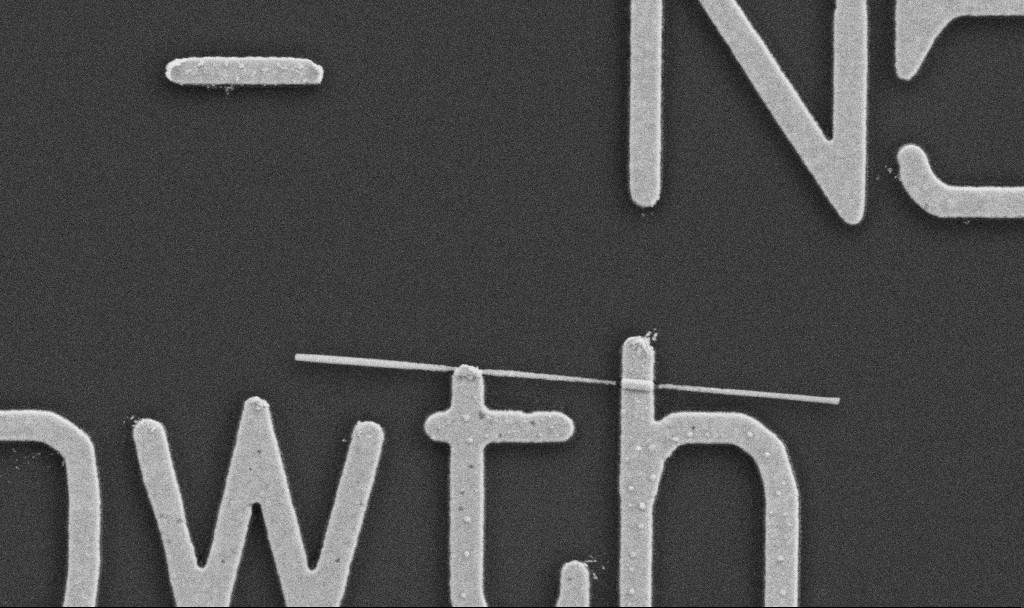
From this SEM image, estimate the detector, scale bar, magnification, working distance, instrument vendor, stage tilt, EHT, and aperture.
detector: SE2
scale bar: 1000 nm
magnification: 30 K X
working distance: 8.7 mm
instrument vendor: Zeiss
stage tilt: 0°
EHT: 5 kV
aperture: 30 µm